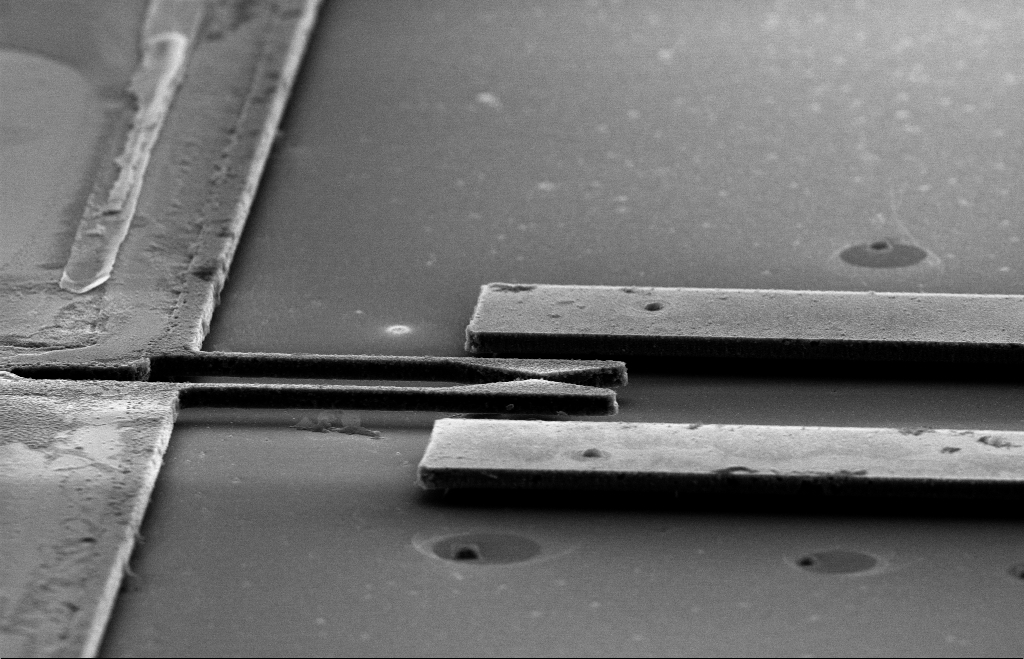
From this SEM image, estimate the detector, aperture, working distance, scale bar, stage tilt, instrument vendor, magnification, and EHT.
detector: SE2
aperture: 30 µm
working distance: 11 mm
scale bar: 10000 nm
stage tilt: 70°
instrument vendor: Zeiss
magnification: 3.2 K X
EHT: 10 kV